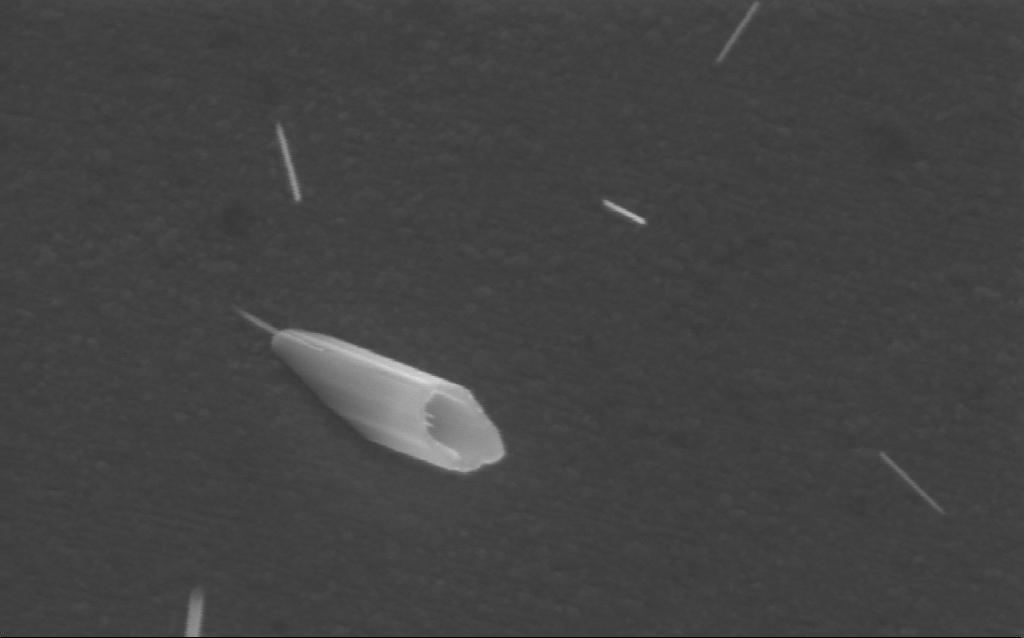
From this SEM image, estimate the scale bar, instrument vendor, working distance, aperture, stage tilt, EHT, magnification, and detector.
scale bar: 200 nm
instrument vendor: Zeiss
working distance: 3 mm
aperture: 30 µm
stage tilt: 23.6°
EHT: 5 kV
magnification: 250.63 K X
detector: InLens